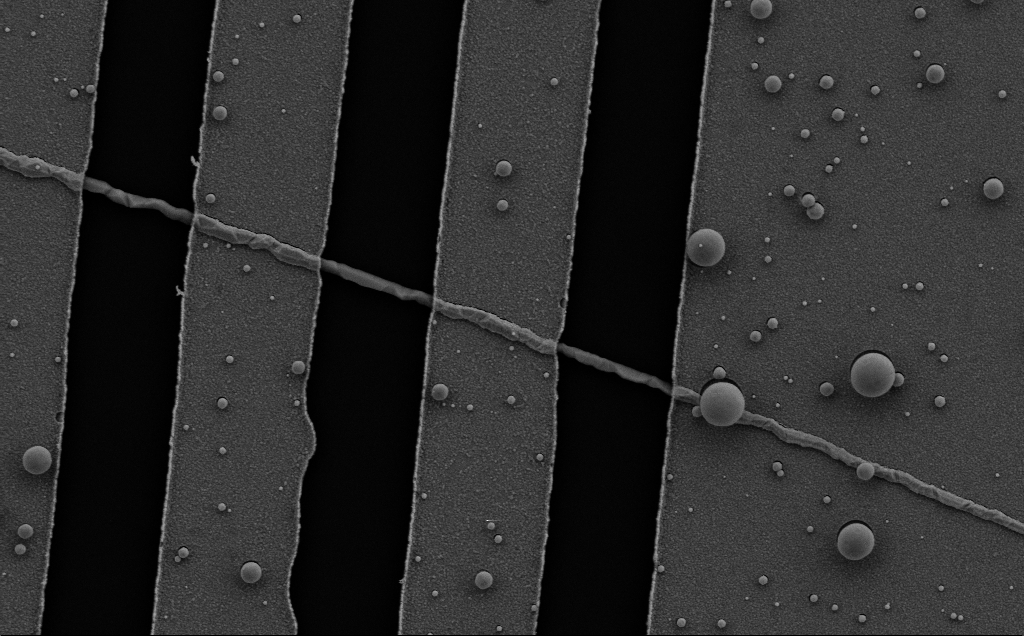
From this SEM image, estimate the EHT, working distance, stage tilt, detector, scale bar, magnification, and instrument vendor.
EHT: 5 kV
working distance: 8 mm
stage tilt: -0.7°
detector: SE2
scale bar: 2000 nm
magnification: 22.77 K X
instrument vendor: Zeiss